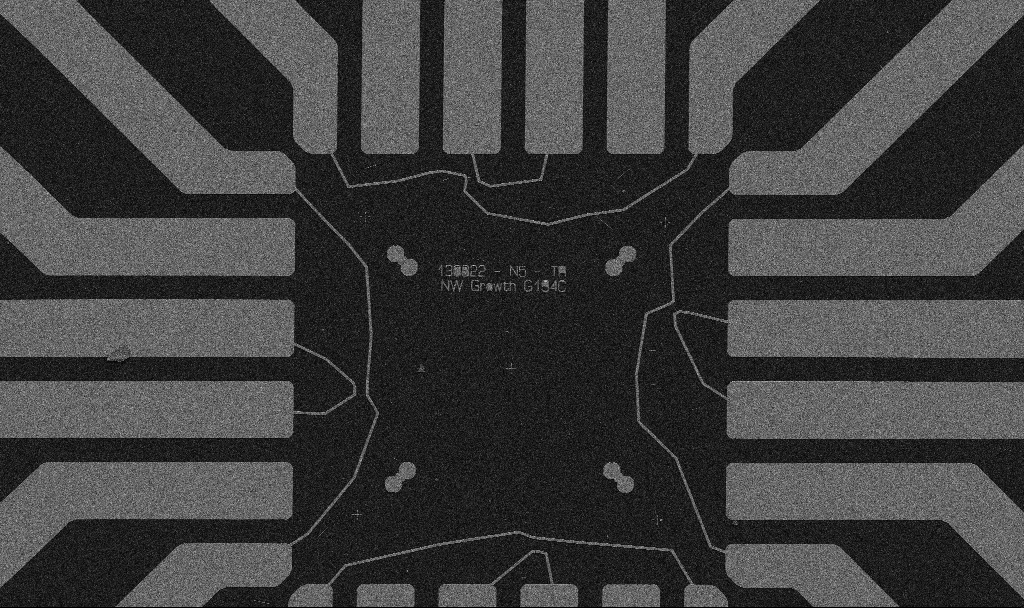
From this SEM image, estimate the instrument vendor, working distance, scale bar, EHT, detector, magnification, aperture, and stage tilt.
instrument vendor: Zeiss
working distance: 10.7 mm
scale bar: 20000 nm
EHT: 5 kV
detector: SE2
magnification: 1 K X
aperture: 30 µm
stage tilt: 0°